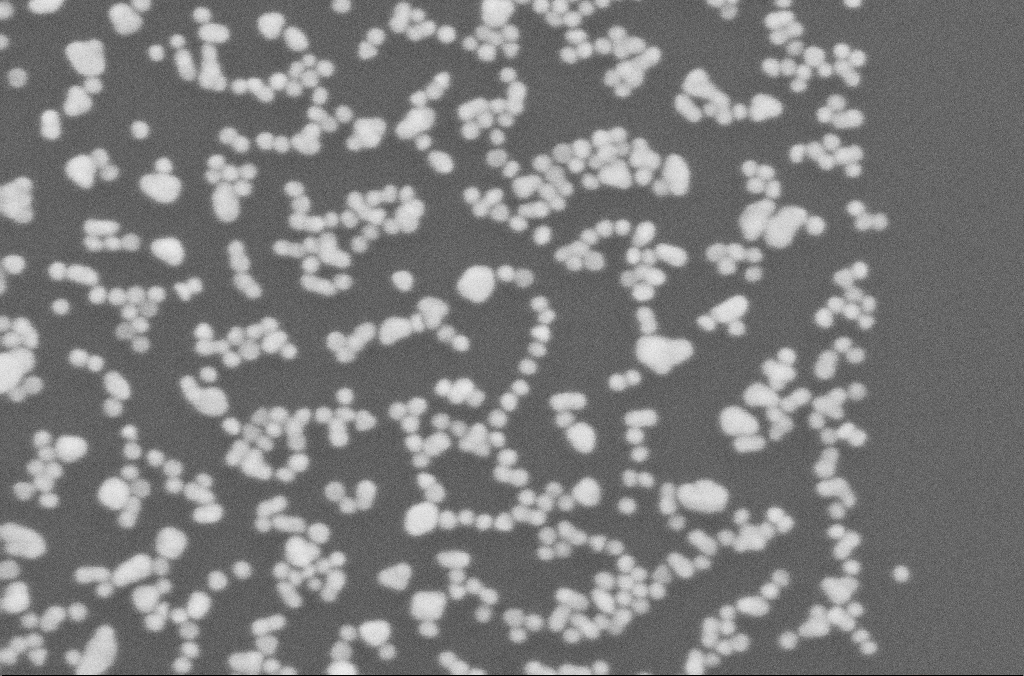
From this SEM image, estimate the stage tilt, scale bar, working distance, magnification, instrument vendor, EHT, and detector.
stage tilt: -0°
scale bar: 100 nm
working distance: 4.9 mm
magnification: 343.68 K X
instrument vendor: Zeiss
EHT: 10 kV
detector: SE2